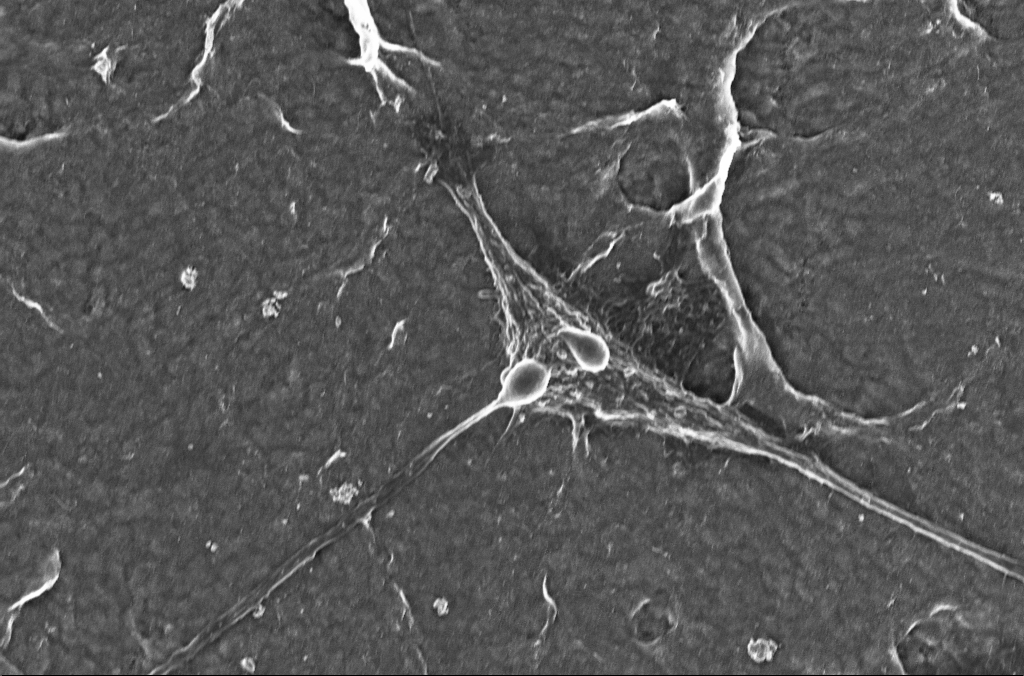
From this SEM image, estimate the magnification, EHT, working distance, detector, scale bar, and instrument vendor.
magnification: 2.5 K X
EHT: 5 kV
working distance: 2.9 mm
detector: InLens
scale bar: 10000 nm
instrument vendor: Zeiss